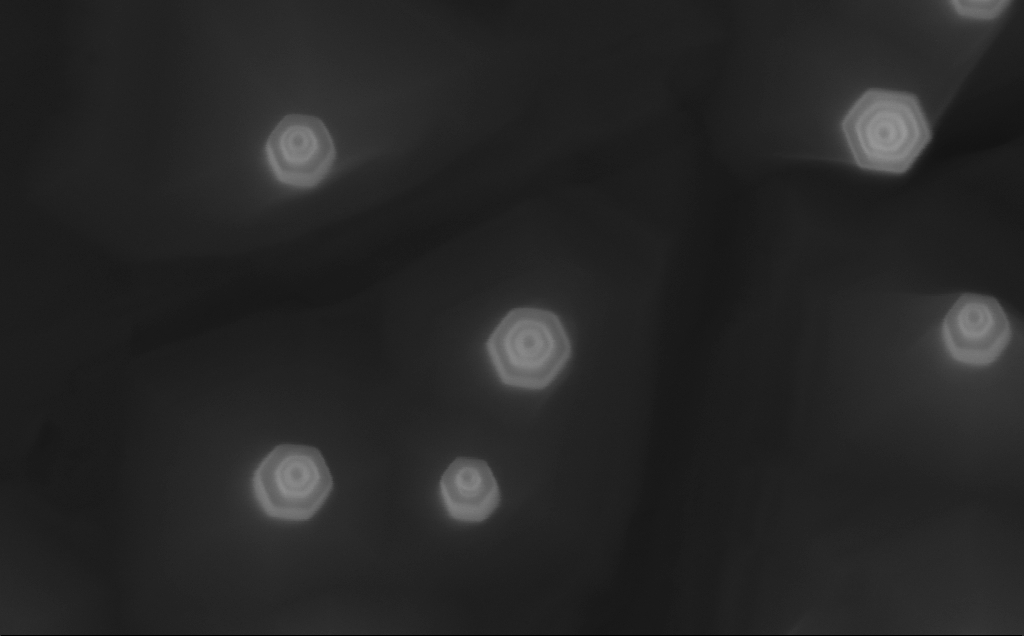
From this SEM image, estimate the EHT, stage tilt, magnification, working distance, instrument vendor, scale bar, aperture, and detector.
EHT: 10 kV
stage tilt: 0°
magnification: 300 K X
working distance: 5 mm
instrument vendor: Zeiss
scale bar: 200 nm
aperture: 30 µm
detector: InLens